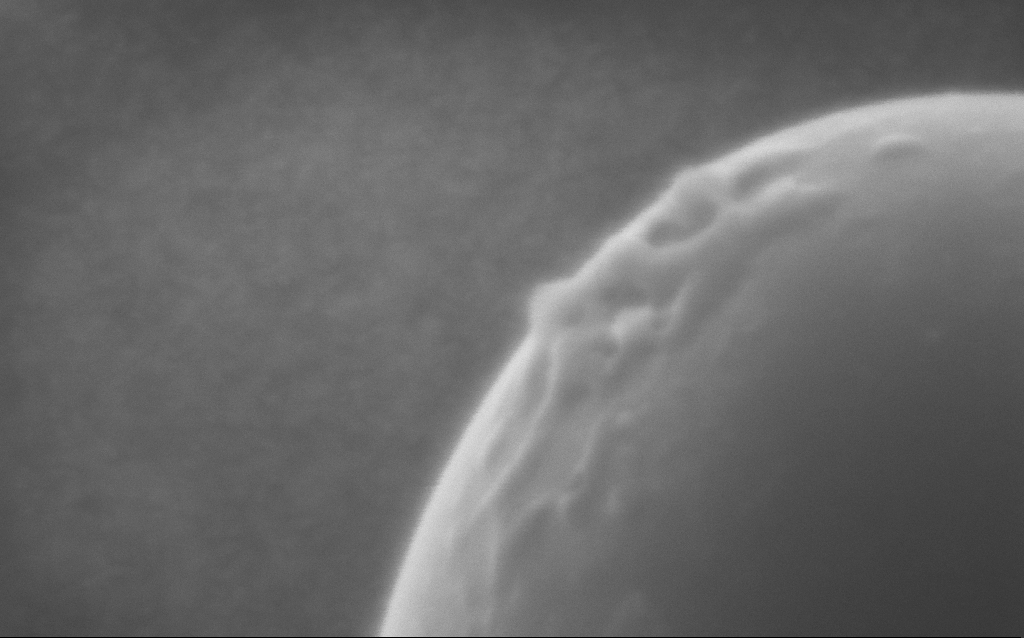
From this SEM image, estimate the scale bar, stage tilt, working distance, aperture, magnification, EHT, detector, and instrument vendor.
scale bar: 100 nm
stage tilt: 0°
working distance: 3 mm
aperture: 30 µm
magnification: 693.1 K X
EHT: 5 kV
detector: InLens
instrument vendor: Zeiss